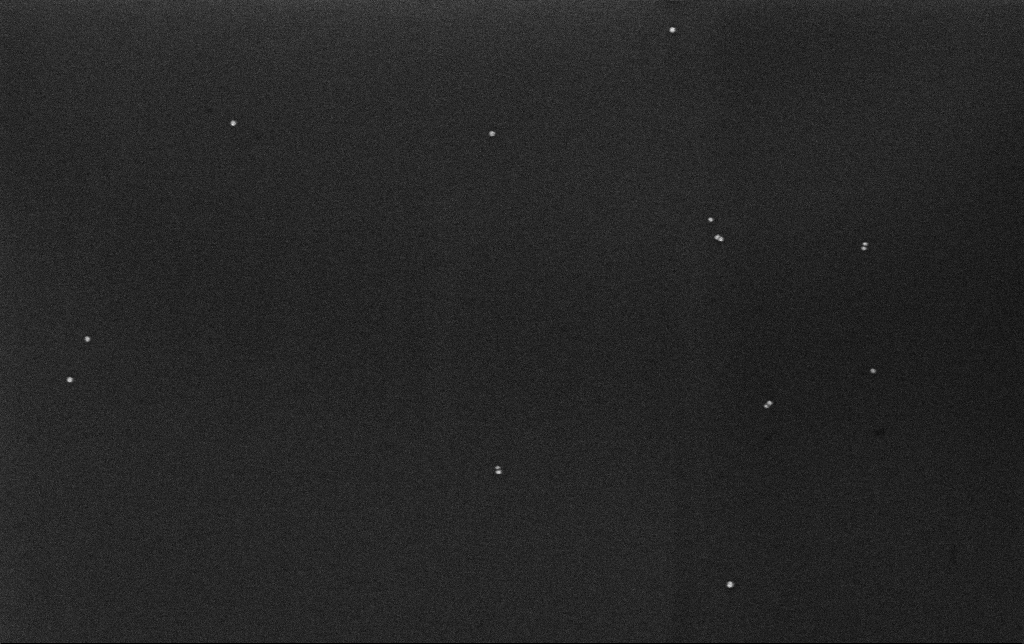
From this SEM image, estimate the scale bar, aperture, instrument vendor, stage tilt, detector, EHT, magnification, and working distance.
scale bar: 200 nm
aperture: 30 µm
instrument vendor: Zeiss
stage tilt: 0°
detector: InLens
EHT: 10 kV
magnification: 100 K X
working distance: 3.2 mm